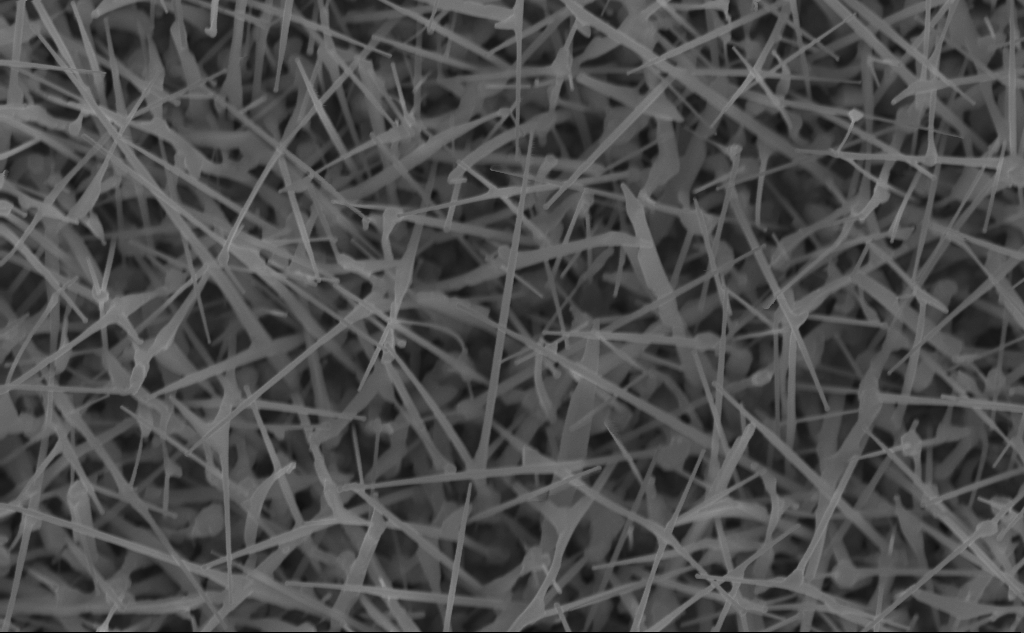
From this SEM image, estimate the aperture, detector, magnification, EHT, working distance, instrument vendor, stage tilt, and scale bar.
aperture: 30 µm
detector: InLens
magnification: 40 K X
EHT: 10 kV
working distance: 5 mm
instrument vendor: Zeiss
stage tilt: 0°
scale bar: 1000 nm